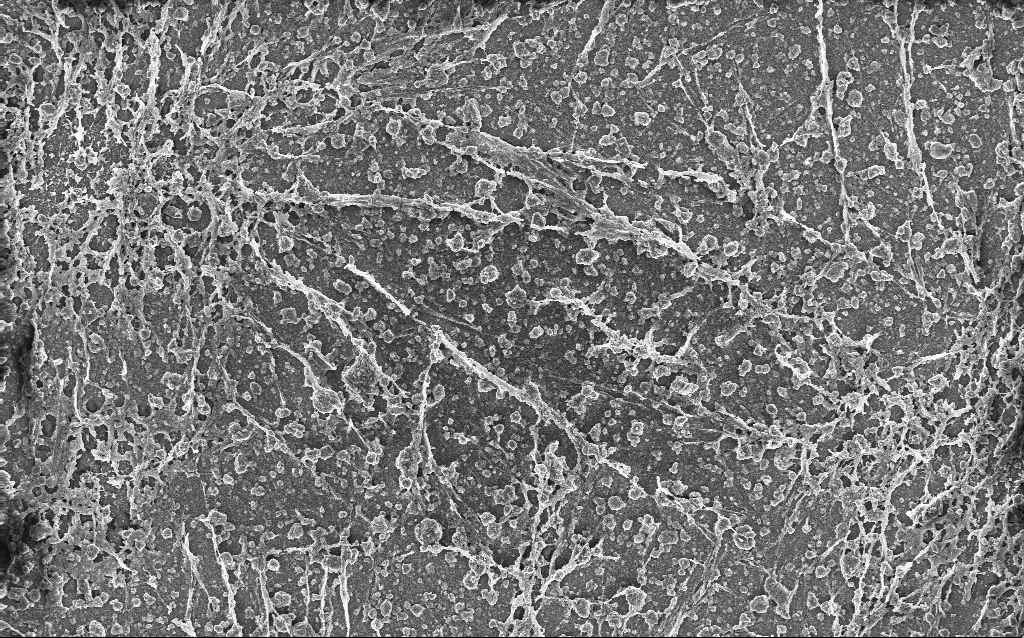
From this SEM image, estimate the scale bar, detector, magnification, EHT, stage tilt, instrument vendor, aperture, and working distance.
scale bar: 20000 nm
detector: InLens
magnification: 0.792 K X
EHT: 10 kV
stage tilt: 0°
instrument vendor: Zeiss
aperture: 30 µm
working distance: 2.7 mm